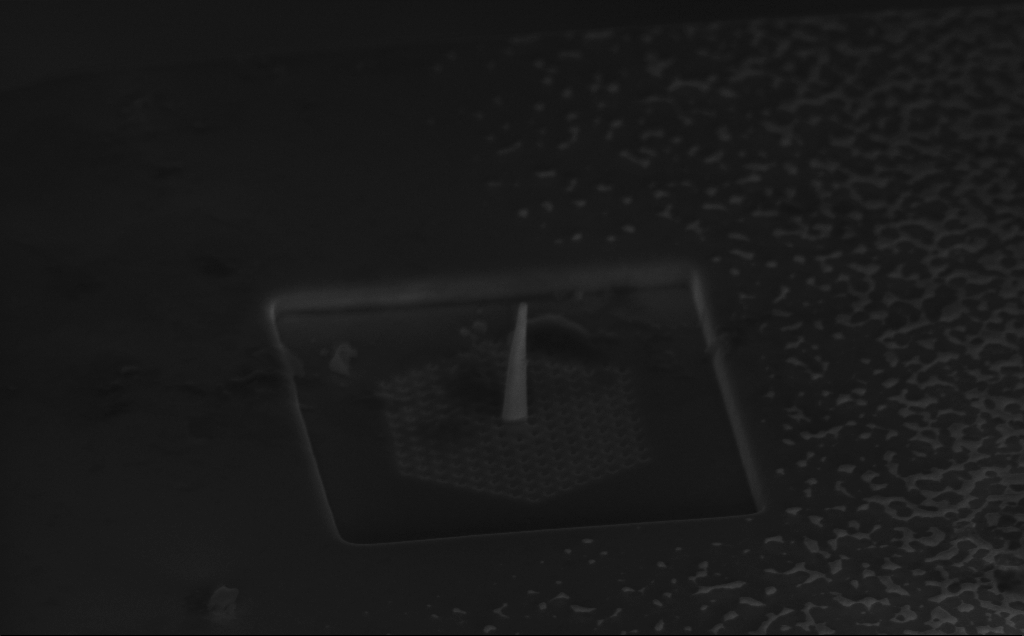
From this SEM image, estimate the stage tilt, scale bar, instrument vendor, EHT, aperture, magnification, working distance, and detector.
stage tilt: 53.9°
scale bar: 1000 nm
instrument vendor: Zeiss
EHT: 5 kV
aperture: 30 µm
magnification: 25.28 K X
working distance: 6 mm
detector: InLens